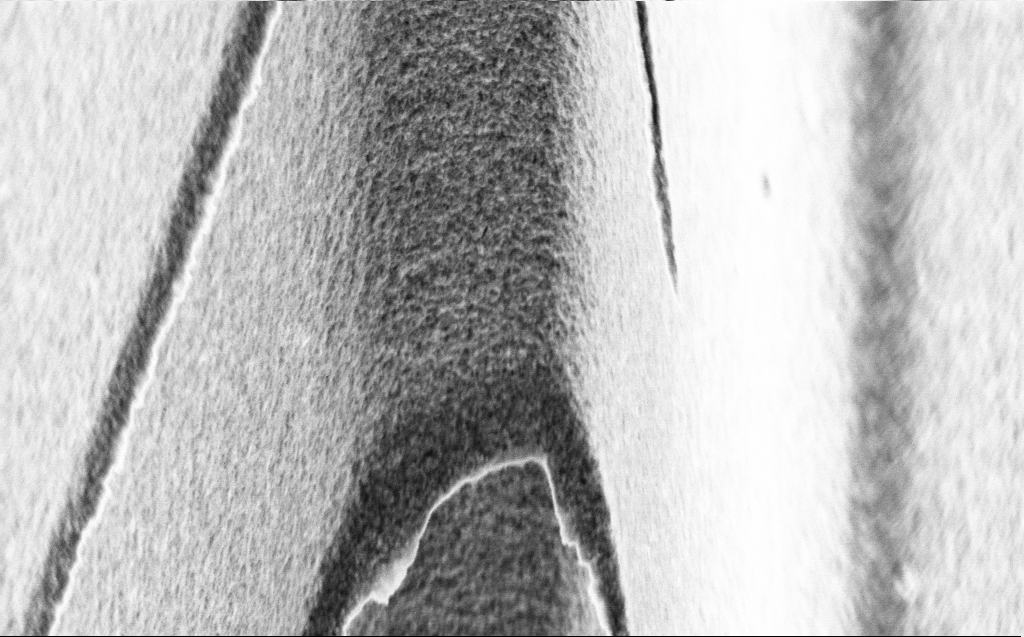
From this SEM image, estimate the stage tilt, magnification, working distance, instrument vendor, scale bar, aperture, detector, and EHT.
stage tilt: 45°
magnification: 39.83 K X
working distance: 4 mm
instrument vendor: Zeiss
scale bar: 1000 nm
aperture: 30 µm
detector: InLens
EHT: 3 kV